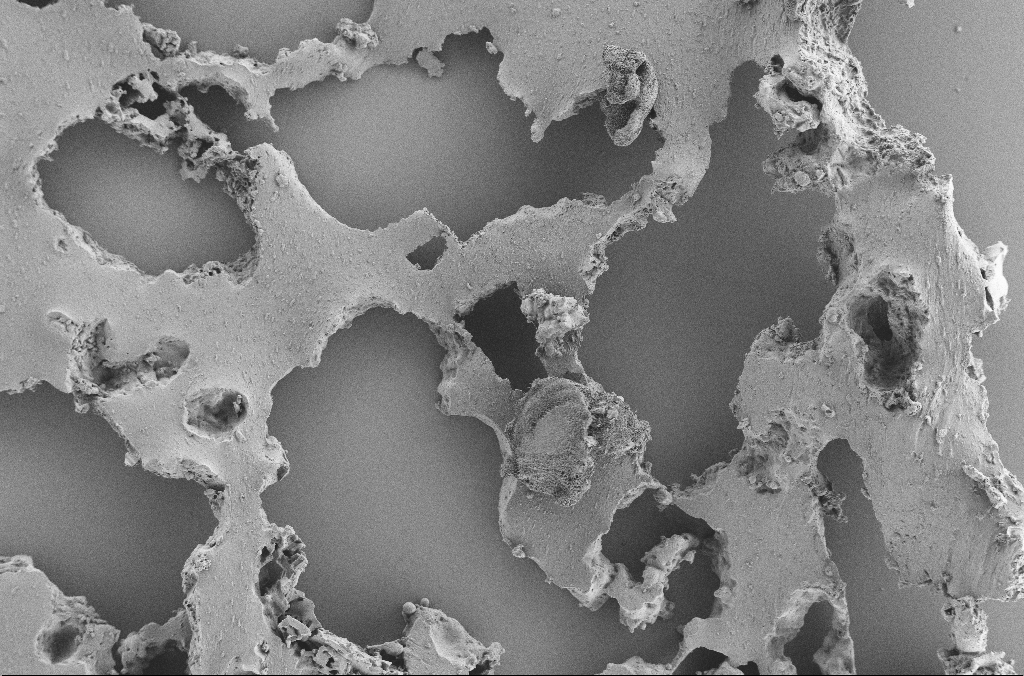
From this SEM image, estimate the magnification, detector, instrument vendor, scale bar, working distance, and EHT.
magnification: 0.25 K X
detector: SE2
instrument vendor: Zeiss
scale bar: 100000 nm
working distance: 3.6 mm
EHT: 2 kV